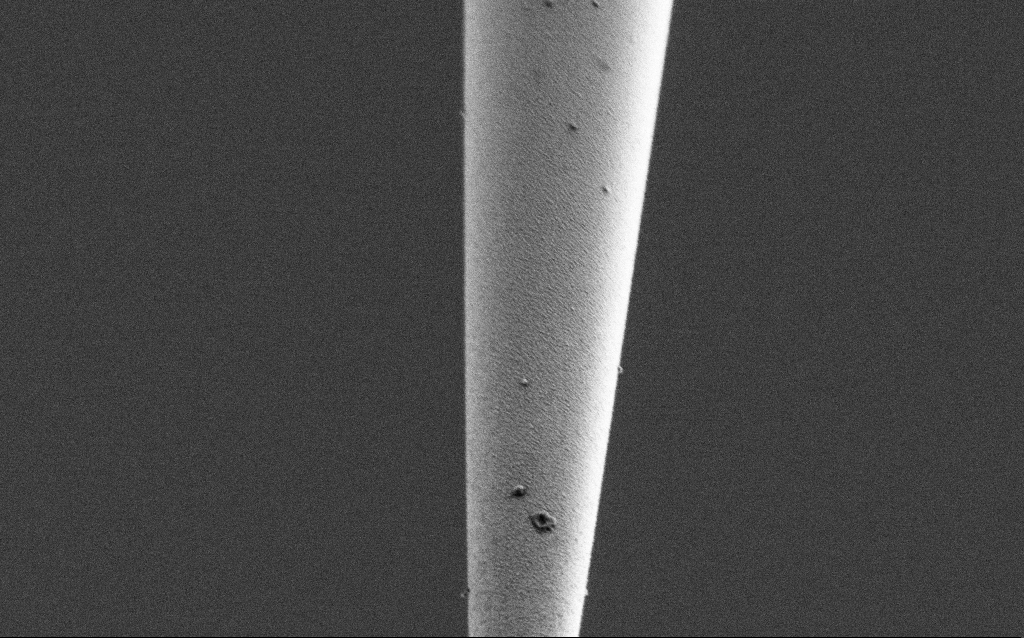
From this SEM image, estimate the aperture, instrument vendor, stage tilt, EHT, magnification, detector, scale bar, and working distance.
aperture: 30 µm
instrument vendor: Zeiss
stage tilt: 45°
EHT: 1 kV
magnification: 25 K X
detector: SE2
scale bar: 2000 nm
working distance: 6.7 mm